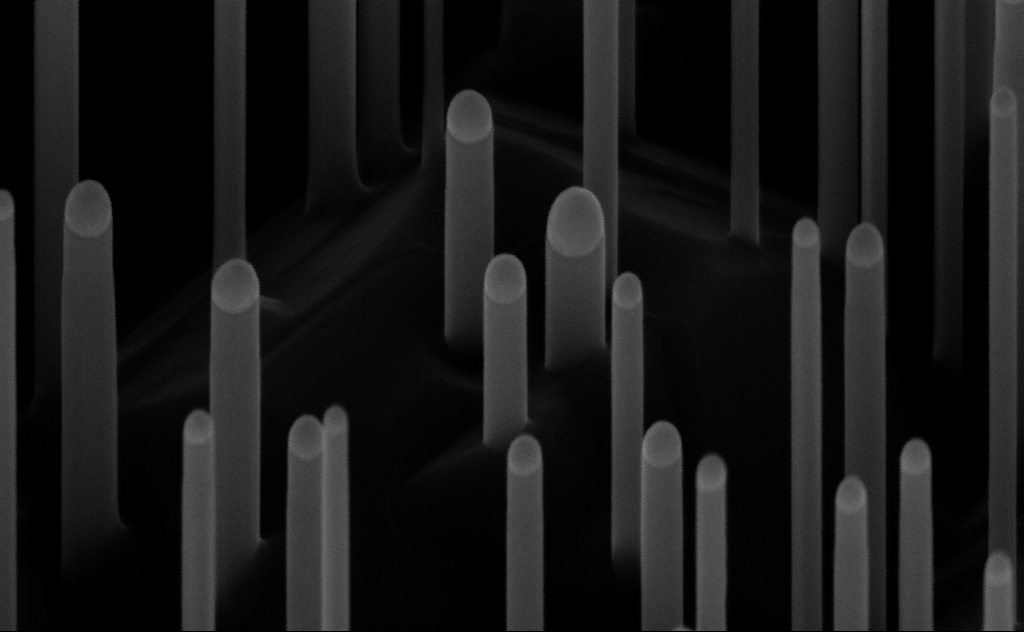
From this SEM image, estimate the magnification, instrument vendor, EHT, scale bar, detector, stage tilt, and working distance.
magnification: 200 K X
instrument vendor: Zeiss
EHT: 10 kV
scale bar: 200 nm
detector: InLens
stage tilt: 45°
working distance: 7 mm